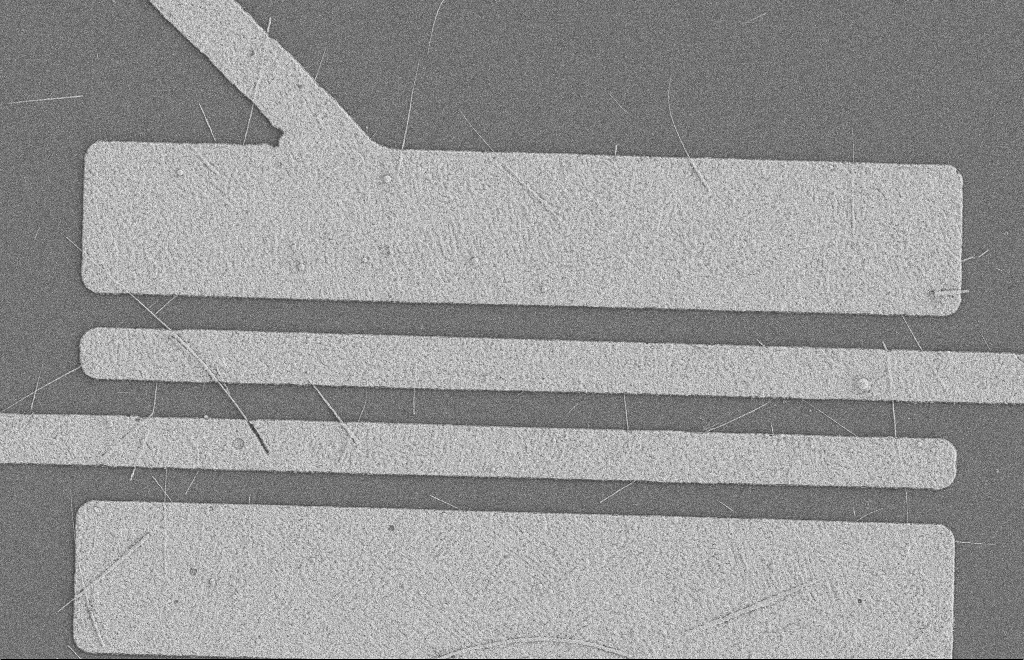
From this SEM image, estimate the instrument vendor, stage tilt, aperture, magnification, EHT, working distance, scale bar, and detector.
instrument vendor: Zeiss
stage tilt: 0°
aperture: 20 µm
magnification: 5.29 K X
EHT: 2 kV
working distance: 8 mm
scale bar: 2000 nm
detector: SE2